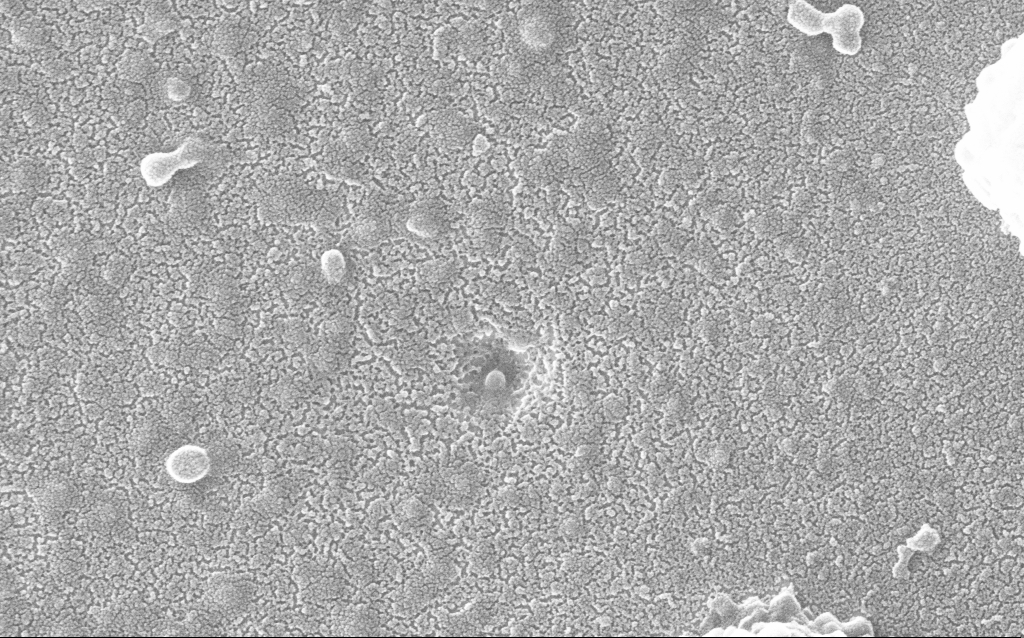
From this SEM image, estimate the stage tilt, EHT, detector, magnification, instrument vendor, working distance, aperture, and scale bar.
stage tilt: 0°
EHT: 20 kV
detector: InLens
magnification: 200 K X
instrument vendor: Zeiss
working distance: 1.5 mm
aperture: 30 µm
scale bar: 100 nm